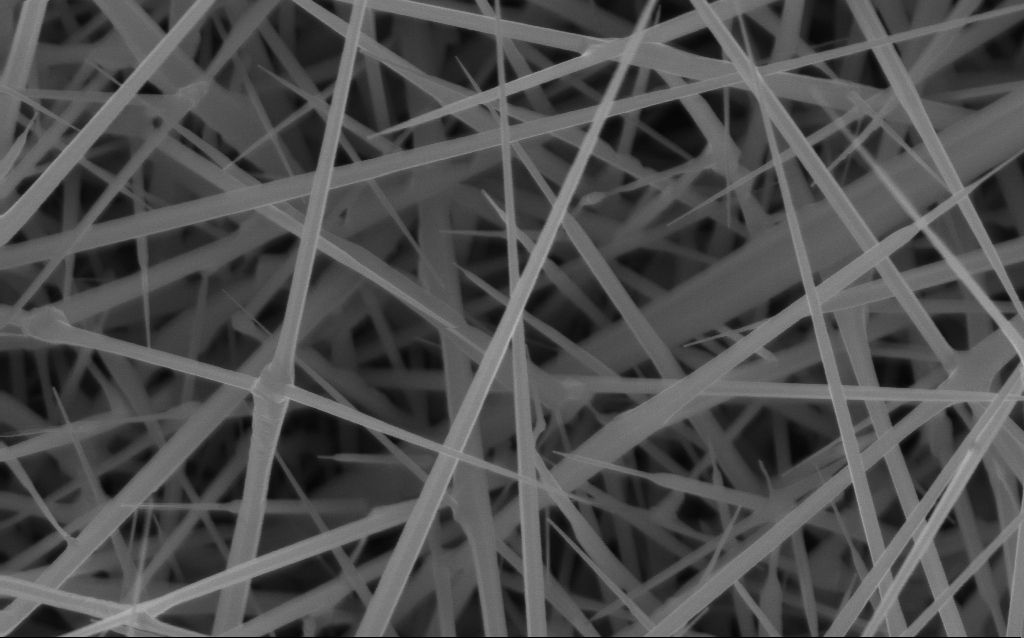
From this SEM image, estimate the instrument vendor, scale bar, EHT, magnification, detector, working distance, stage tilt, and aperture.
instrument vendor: Zeiss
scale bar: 1000 nm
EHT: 10 kV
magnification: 40 K X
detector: InLens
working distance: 4 mm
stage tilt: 0°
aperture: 30 µm